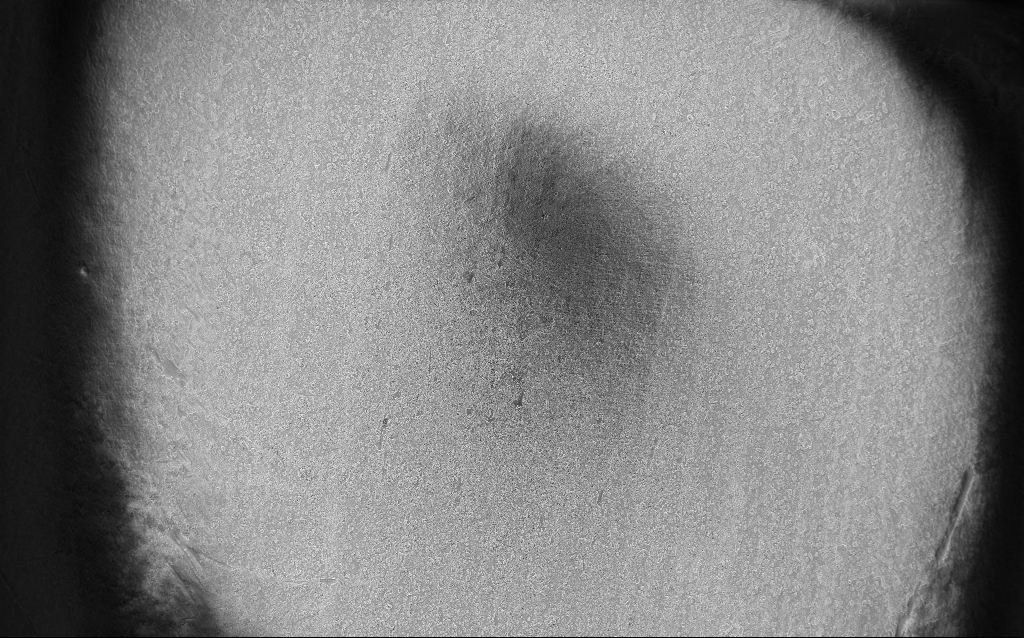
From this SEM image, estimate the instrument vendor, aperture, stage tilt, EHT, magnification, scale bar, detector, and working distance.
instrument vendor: Zeiss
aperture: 30 µm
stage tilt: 0°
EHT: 5 kV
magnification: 0.11 K X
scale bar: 200000 nm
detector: InLens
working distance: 2.5 mm